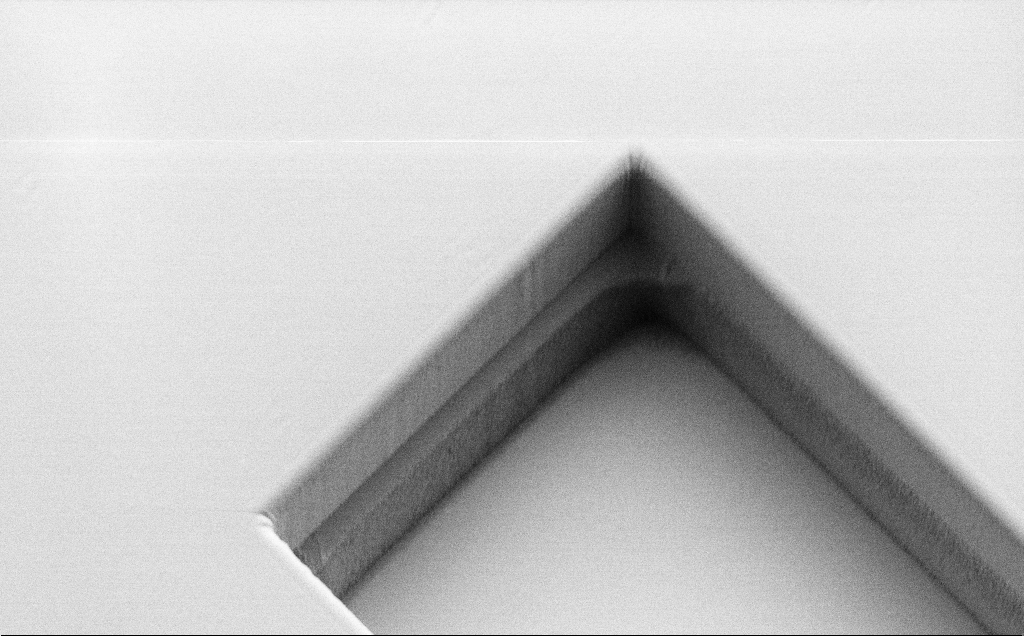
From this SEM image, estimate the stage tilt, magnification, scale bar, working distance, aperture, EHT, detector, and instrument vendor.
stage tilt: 45°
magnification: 2.24 K X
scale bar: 20000 nm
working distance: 8 mm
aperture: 30 µm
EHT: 1.3 kV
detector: SE2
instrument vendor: Zeiss